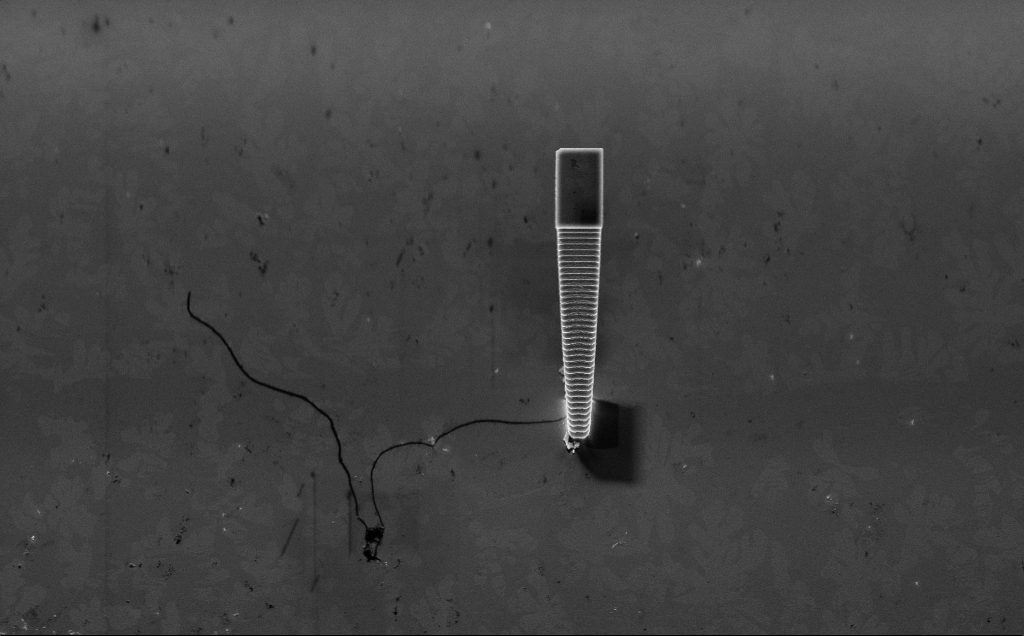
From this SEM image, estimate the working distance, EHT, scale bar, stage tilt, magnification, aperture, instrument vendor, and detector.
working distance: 7 mm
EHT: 5 kV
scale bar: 10000 nm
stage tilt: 45°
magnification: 6.02 K X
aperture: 30 µm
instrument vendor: Zeiss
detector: InLens